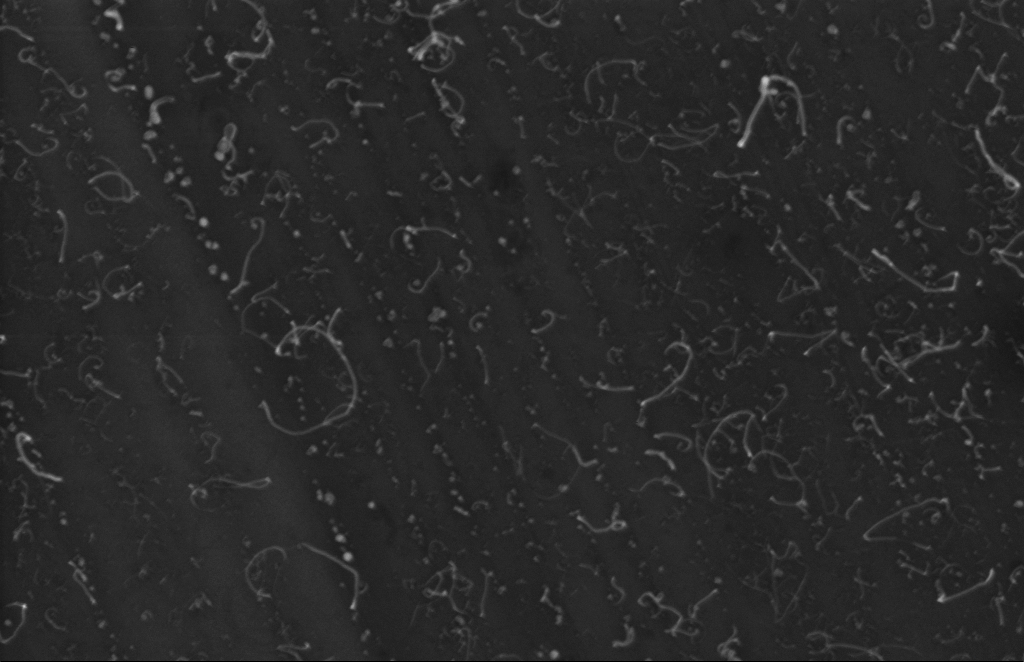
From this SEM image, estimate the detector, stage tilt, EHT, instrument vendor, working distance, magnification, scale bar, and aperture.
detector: InLens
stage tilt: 0°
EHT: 5 kV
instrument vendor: Zeiss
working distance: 5 mm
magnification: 80.85 K X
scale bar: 200 nm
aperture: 20 µm